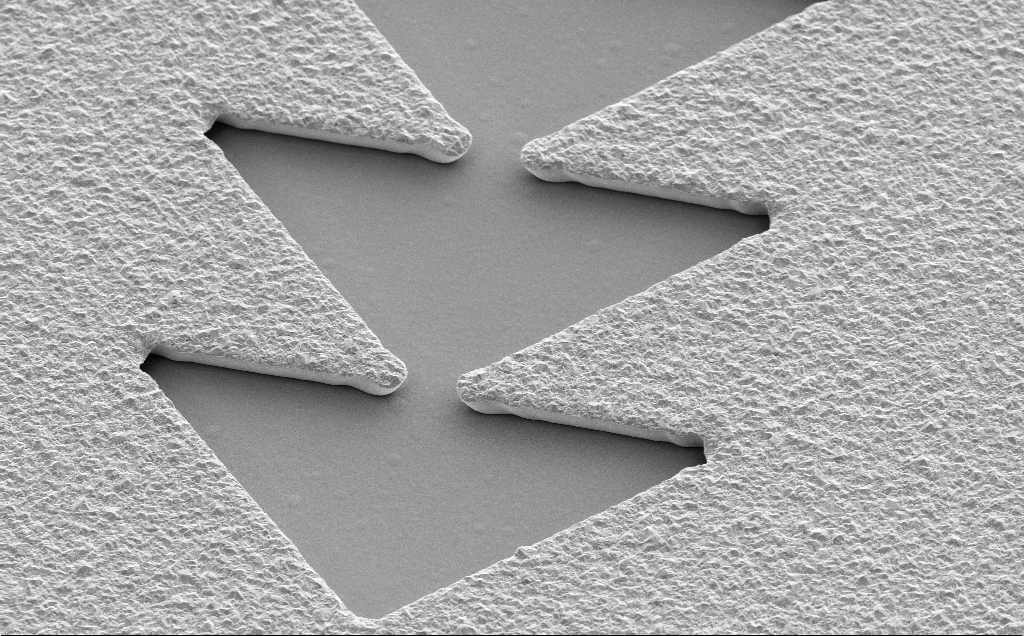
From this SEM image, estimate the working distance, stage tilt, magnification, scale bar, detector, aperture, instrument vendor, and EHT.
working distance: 13 mm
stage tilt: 35°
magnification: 5.93 K X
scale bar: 10000 nm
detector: SE2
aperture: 30 µm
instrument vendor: Zeiss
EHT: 5 kV